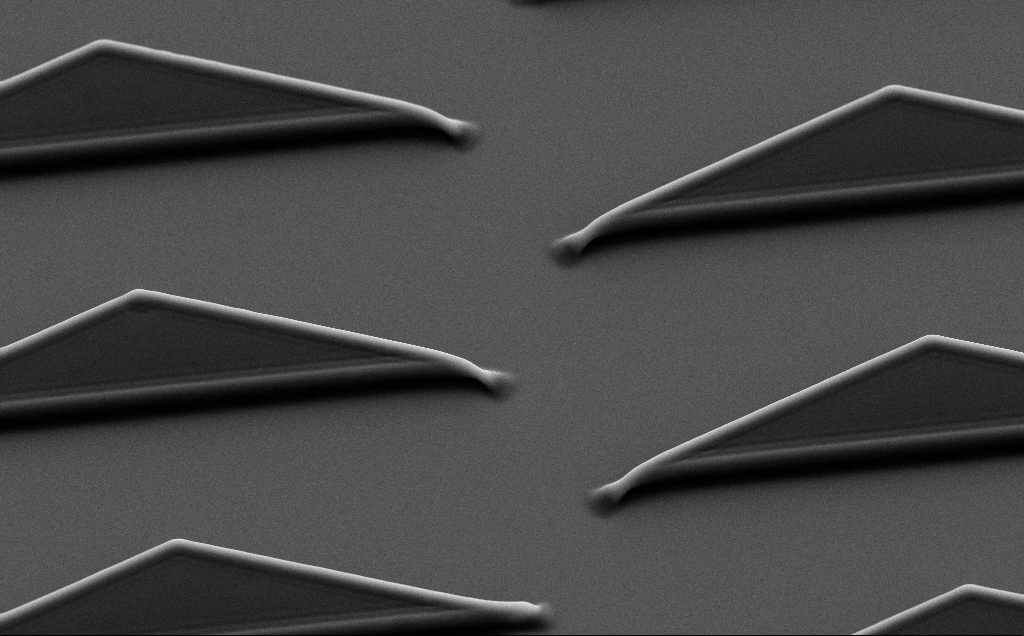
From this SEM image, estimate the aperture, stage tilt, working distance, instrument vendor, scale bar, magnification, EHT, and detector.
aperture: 30 µm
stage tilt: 35°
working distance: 6 mm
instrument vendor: Zeiss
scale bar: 10000 nm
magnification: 6.78 K X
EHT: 7 kV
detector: SE2